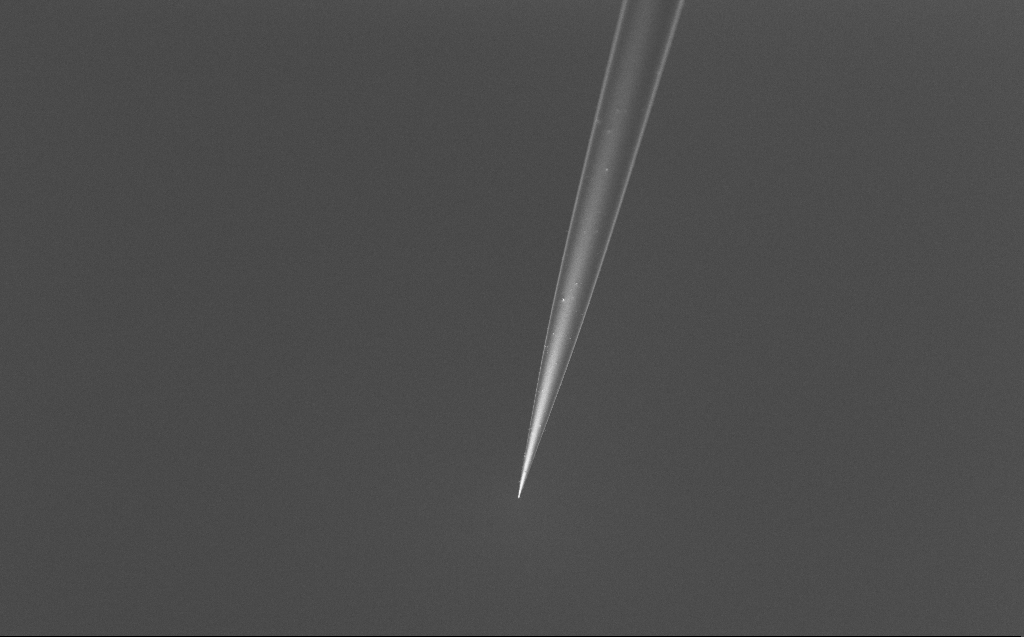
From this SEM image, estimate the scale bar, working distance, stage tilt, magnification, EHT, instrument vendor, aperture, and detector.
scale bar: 20000 nm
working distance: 6 mm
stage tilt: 45°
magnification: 0.828 K X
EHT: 2 kV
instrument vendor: Zeiss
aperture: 30 µm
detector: InLens